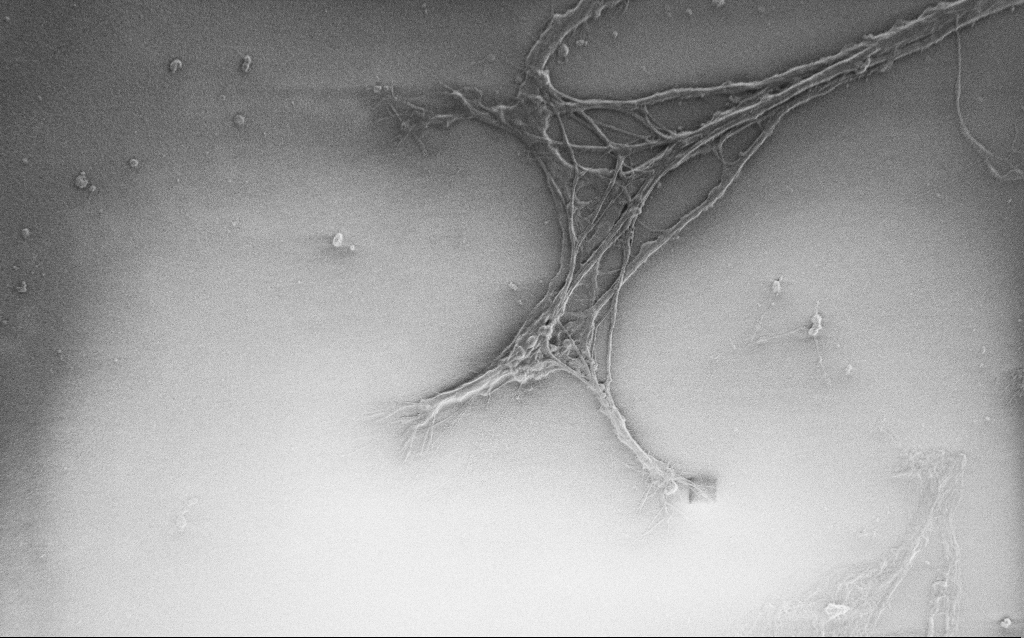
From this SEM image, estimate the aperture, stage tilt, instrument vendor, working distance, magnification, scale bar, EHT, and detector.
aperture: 30 µm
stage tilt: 0°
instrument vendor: Zeiss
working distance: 6 mm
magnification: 2.5 K X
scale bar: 20000 nm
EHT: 0.9 kV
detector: SE2